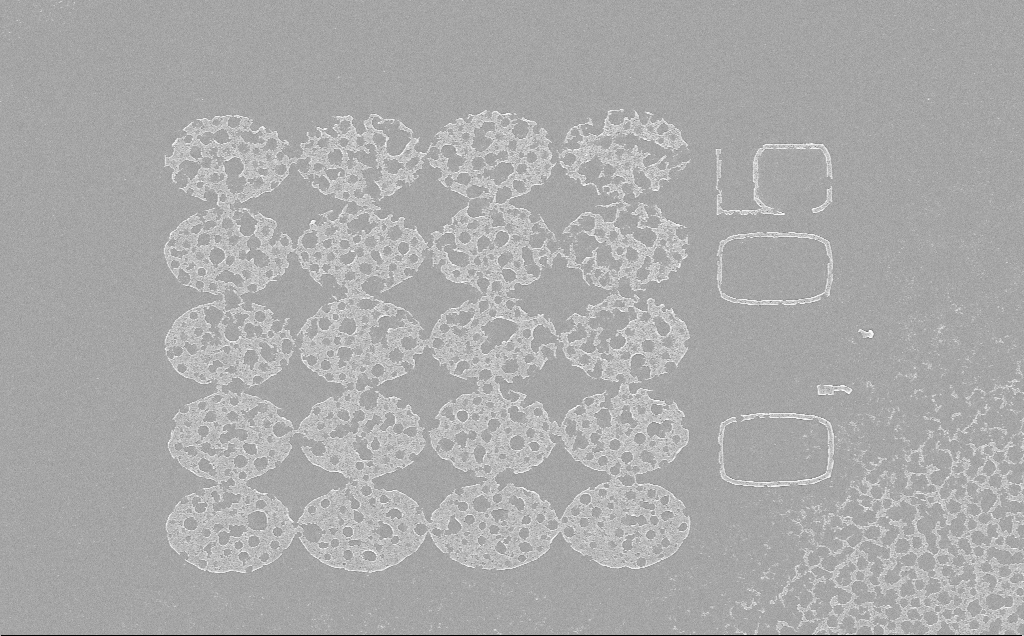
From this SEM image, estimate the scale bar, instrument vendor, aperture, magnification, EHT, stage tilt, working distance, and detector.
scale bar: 10000 nm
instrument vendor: Zeiss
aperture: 30 µm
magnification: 4.87 K X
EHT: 5 kV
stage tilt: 0°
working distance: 6 mm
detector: InLens